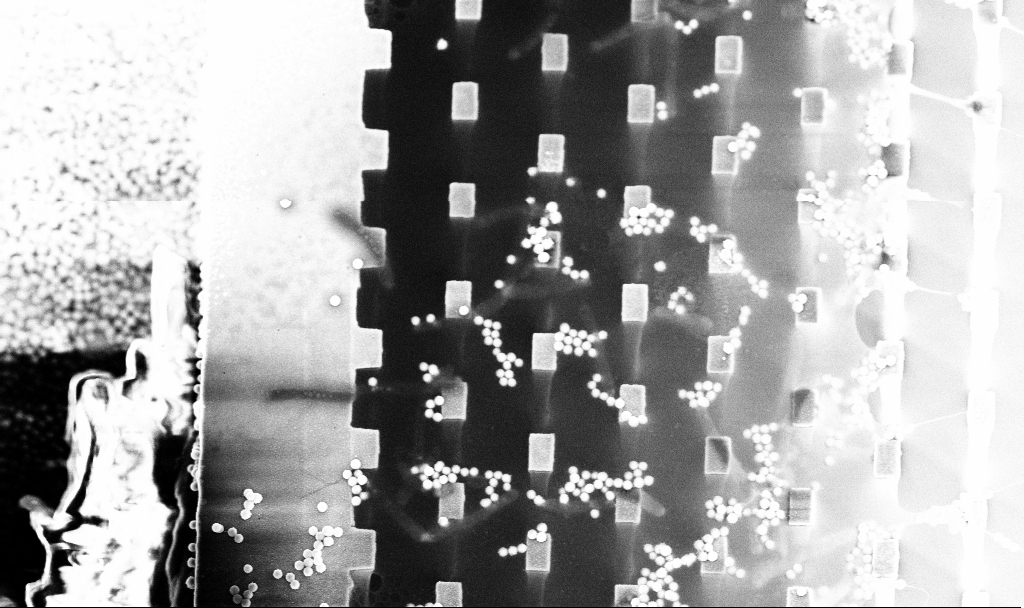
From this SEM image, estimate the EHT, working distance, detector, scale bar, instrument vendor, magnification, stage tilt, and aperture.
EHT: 5 kV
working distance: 3.2 mm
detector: InLens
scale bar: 10000 nm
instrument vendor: Zeiss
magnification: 5.91 K X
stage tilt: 15°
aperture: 30 µm